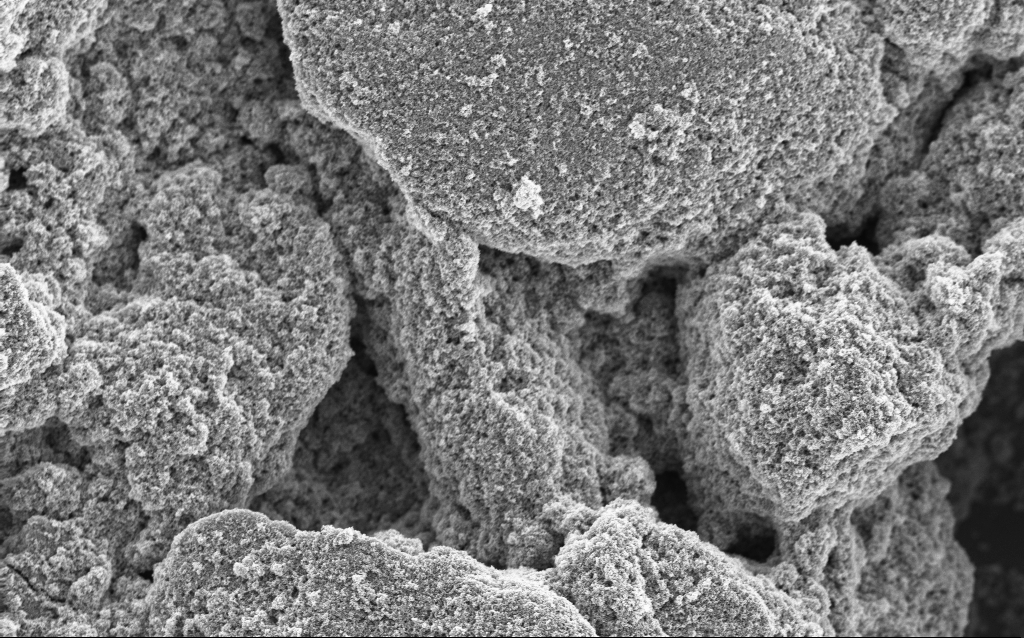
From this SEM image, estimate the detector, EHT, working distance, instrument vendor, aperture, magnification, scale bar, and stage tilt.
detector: InLens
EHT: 5 kV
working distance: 4.5 mm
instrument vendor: Zeiss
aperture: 30 µm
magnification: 6.4 K X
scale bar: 10000 nm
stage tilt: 0°